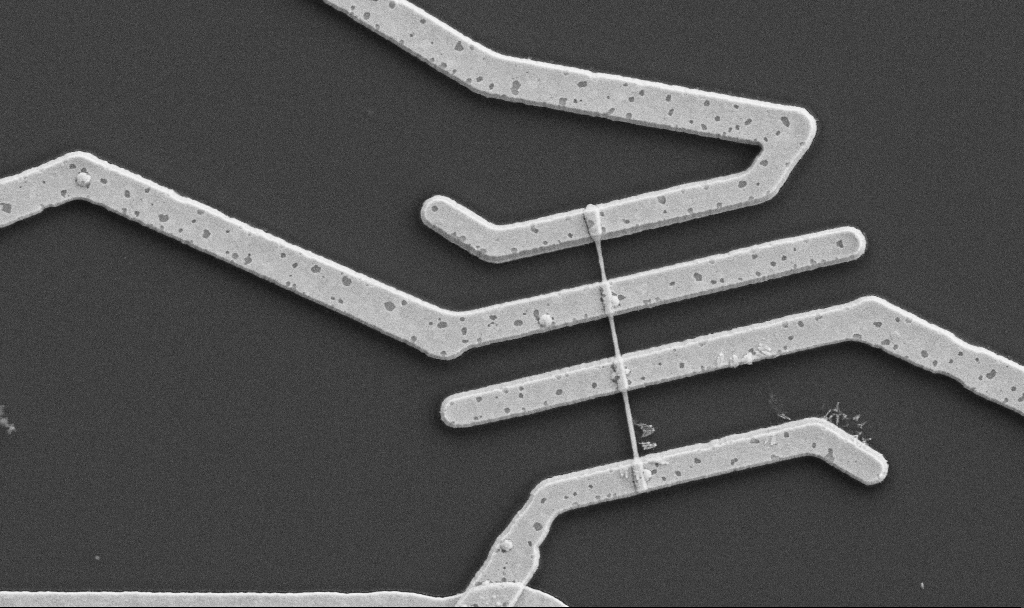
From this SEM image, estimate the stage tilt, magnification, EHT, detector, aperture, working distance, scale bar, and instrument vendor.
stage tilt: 0°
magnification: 20 K X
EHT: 5 kV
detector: SE2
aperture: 30 µm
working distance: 10.7 mm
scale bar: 1000 nm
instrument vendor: Zeiss